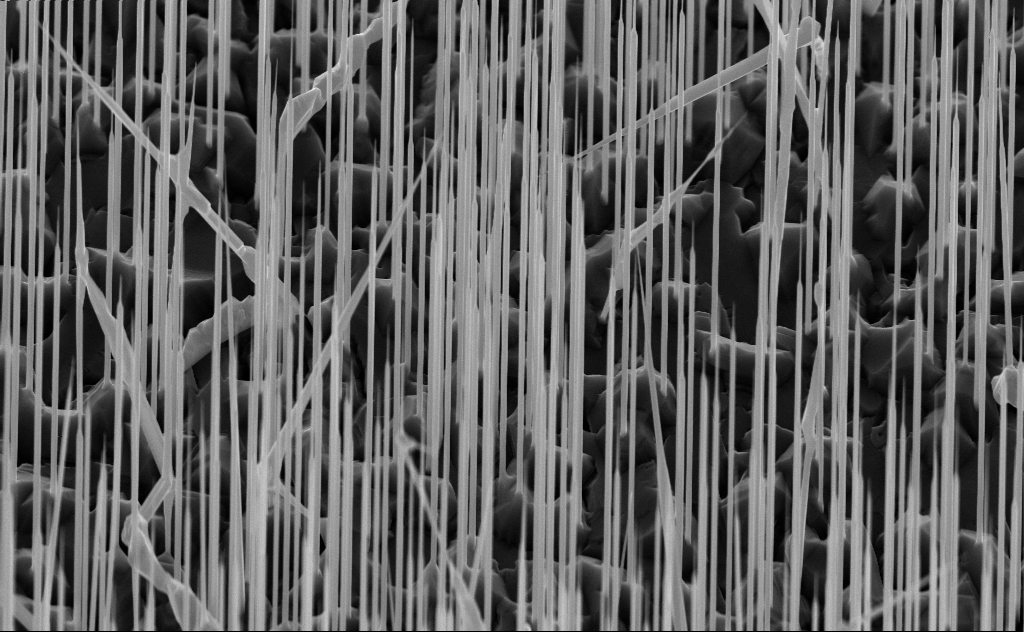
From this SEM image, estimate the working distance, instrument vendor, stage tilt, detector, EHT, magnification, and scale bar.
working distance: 6 mm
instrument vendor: Zeiss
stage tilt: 45°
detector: InLens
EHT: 10 kV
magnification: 20 K X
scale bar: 2000 nm